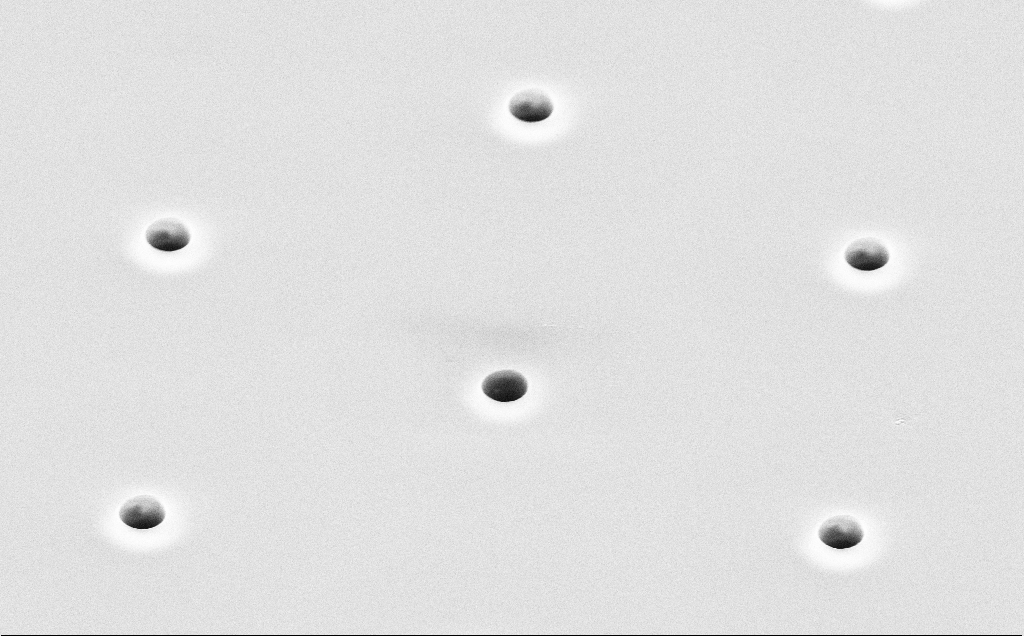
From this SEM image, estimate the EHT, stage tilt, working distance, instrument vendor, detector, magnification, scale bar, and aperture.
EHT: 10 kV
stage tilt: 45°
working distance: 13 mm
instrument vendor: Zeiss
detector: SE2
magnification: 7.23 K X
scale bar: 2000 nm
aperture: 30 µm